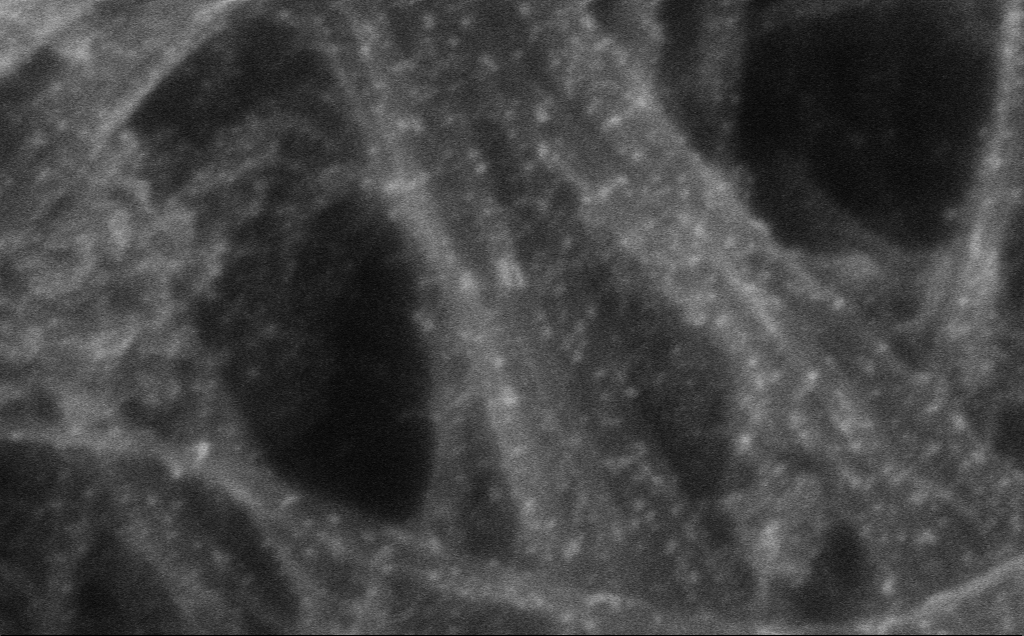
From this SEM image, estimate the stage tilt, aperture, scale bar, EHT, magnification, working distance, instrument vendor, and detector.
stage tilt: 0°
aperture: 30 µm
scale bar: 20 nm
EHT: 10 kV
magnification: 790.09 K X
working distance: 3 mm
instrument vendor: Zeiss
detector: InLens